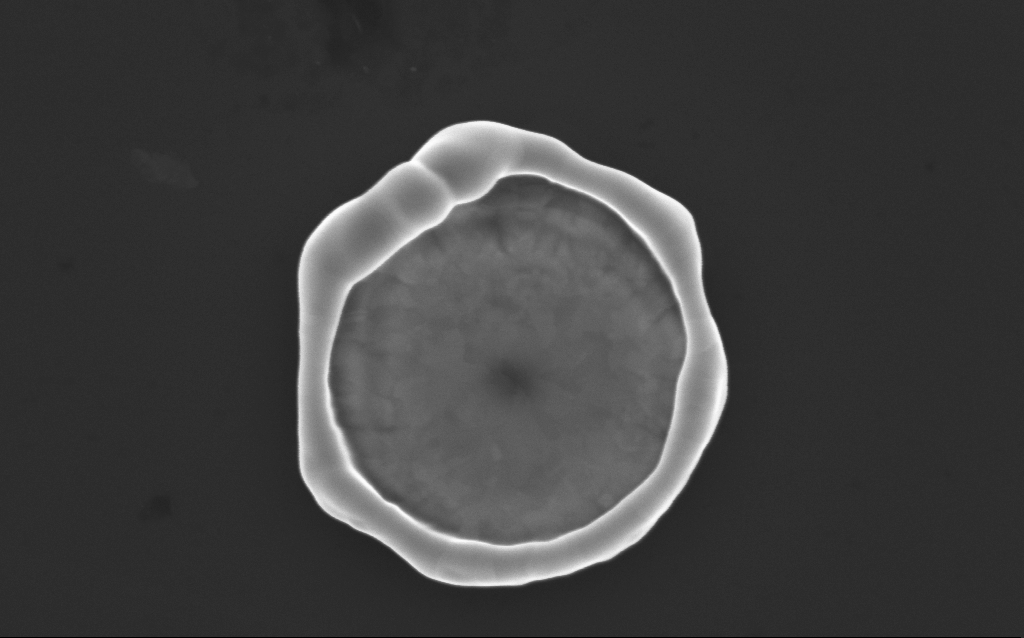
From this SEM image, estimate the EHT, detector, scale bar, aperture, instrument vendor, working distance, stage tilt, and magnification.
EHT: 10 kV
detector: InLens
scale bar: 1000 nm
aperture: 30 µm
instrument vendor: Zeiss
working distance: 4 mm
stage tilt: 0°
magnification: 58.55 K X